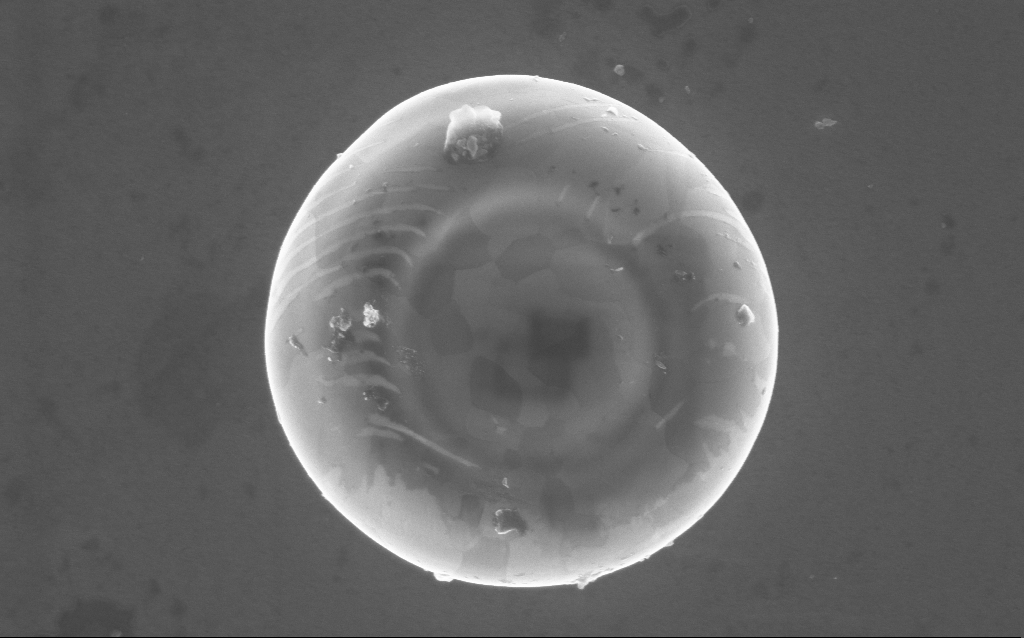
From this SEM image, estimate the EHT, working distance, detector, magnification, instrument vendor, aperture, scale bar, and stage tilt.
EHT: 5 kV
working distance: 4 mm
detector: InLens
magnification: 50 K X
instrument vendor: Zeiss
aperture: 30 µm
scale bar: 1000 nm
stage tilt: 0°